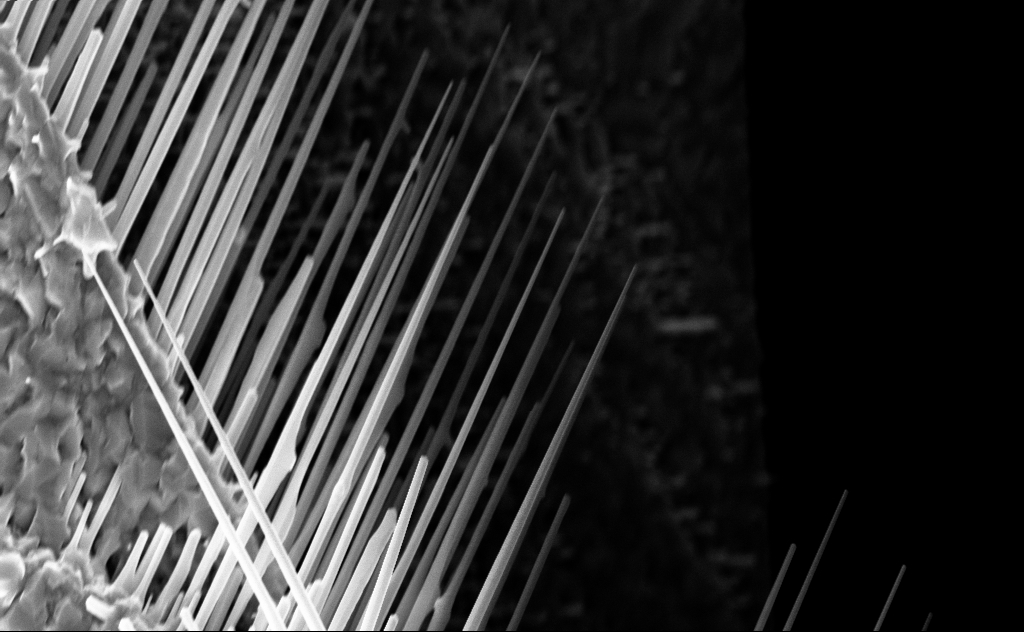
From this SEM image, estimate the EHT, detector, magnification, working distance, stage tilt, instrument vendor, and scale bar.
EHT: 10 kV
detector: InLens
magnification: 20 K X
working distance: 8 mm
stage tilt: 0°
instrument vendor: Zeiss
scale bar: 2000 nm